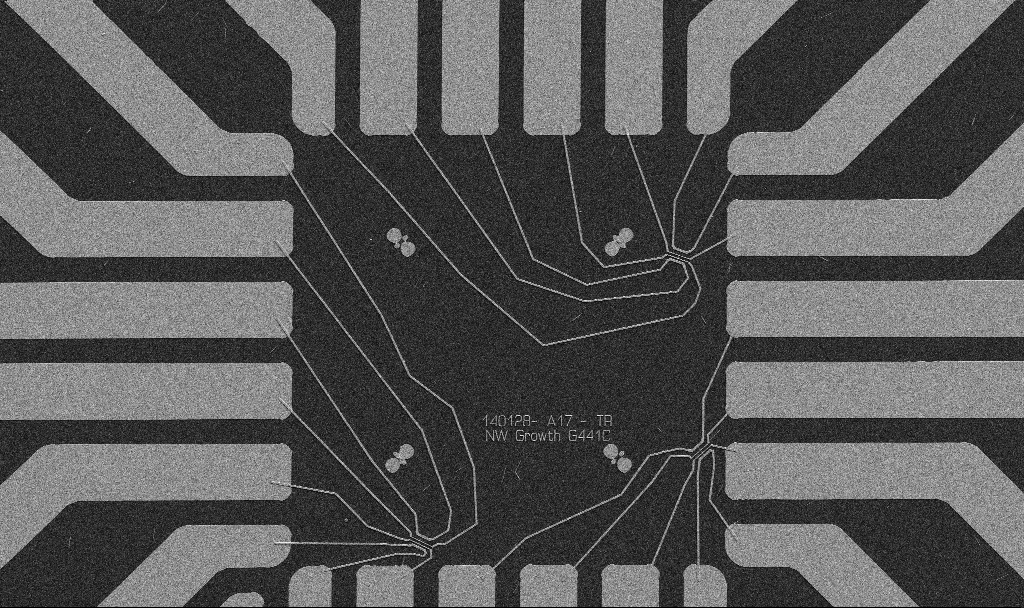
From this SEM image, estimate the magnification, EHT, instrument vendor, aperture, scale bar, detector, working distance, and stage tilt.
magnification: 1 K X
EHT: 5 kV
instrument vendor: Zeiss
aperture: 30 µm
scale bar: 20000 nm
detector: SE2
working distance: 10.7 mm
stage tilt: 0°